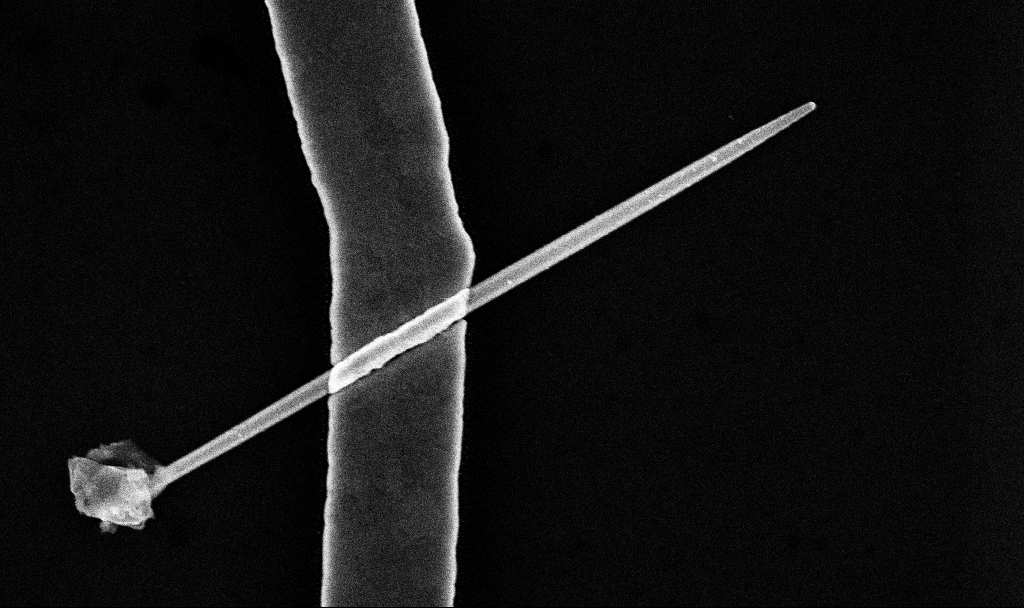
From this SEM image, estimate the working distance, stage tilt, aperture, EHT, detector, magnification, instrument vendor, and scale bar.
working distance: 7.7 mm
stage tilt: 0°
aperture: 30 µm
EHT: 10 kV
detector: InLens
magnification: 56.96 K X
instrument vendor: Zeiss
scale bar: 1000 nm